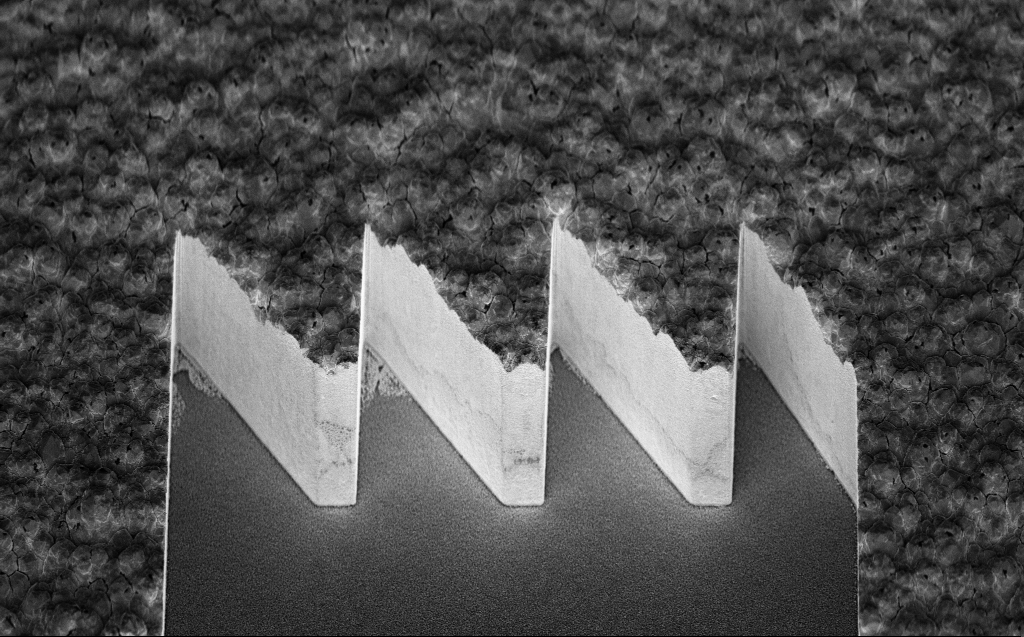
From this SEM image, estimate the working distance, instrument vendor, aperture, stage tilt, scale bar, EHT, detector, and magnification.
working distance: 6 mm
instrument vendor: Zeiss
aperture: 30 µm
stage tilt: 45°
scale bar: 10000 nm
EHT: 5 kV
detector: InLens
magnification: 6.09 K X